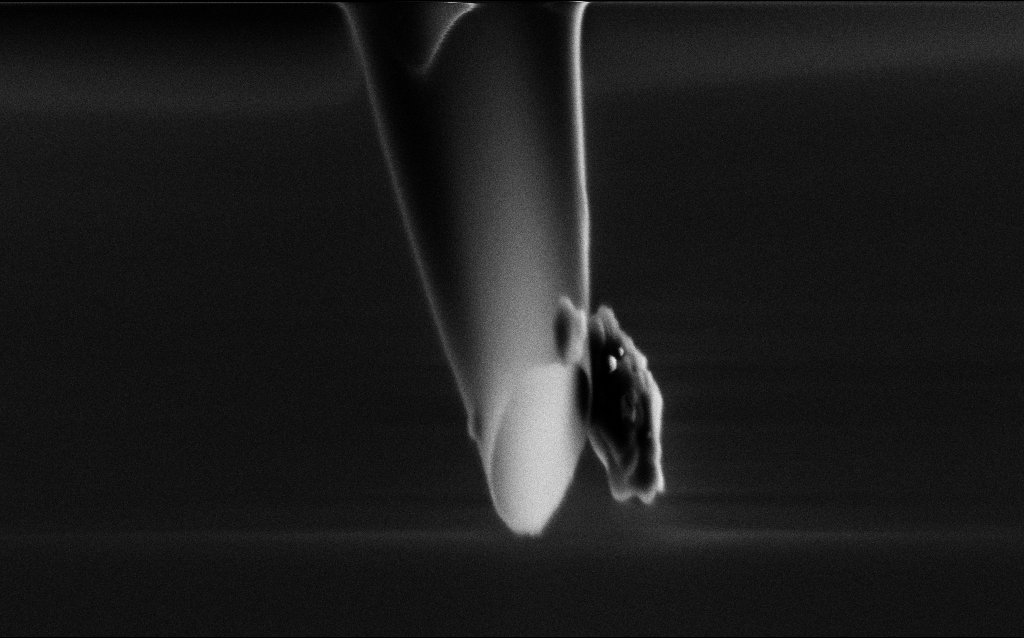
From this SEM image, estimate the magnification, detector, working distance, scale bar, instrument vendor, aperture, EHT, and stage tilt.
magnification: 65.84 K X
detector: SE2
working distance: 5 mm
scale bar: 1000 nm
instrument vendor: Zeiss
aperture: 30 µm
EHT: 2 kV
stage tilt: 45°